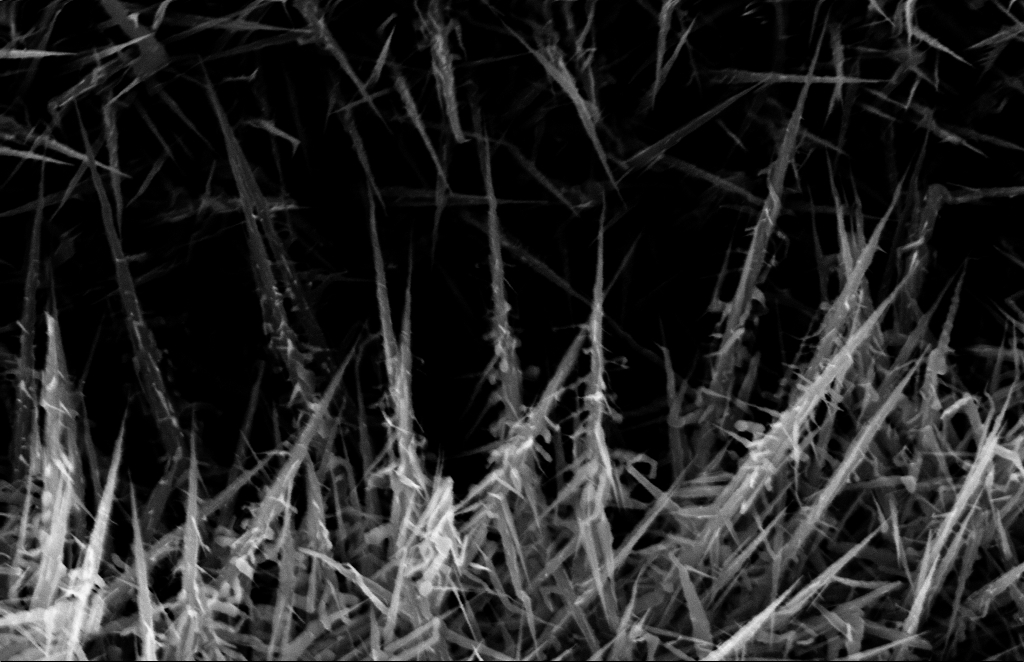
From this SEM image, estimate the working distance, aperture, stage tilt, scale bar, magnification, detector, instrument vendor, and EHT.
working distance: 10 mm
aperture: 30 µm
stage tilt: -1.1°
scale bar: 200 nm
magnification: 64.09 K X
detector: InLens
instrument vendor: Zeiss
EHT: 10 kV